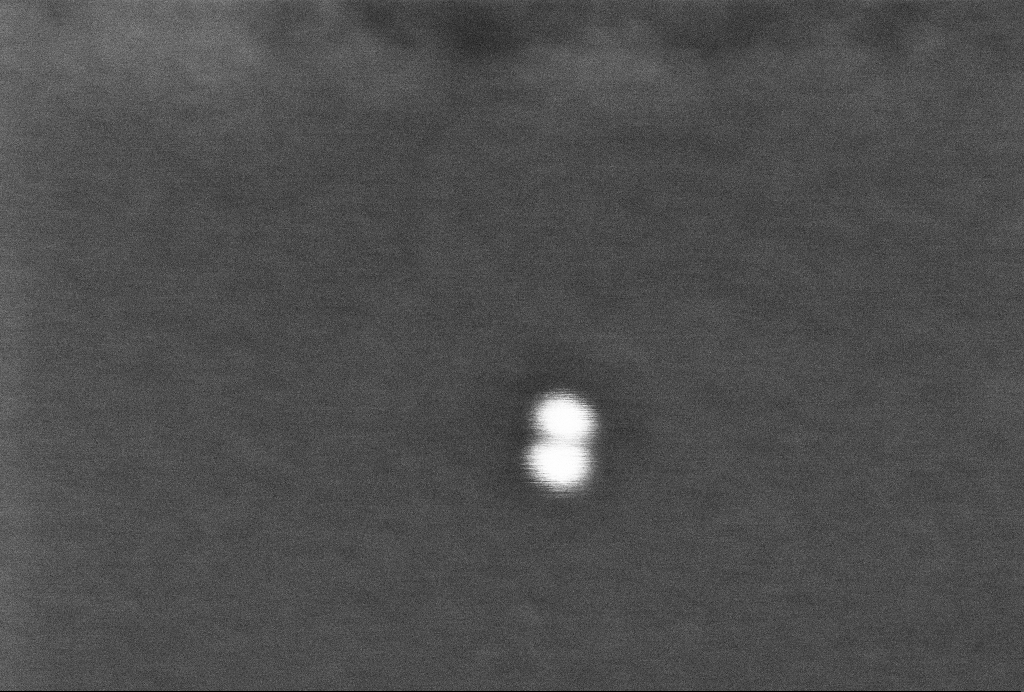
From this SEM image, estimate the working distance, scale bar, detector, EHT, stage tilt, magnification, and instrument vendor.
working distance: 3.3 mm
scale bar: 20 nm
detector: InLens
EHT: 2 kV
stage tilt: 0°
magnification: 717.1 K X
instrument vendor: Zeiss